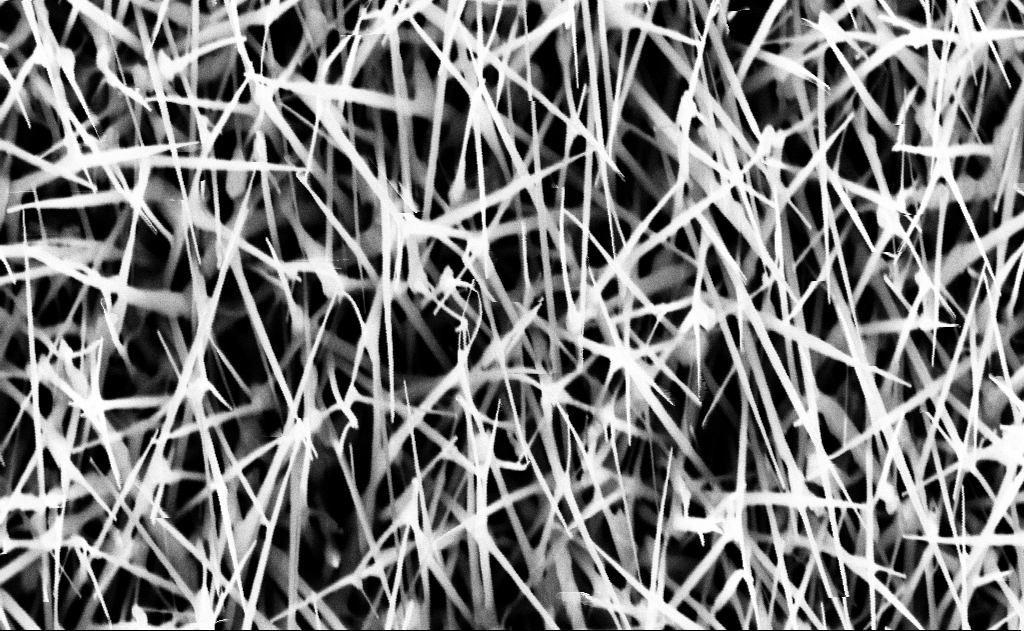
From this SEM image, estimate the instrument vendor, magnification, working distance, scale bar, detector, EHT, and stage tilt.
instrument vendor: Zeiss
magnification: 40 K X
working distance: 16 mm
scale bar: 1000 nm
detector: InLens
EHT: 10 kV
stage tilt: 0°